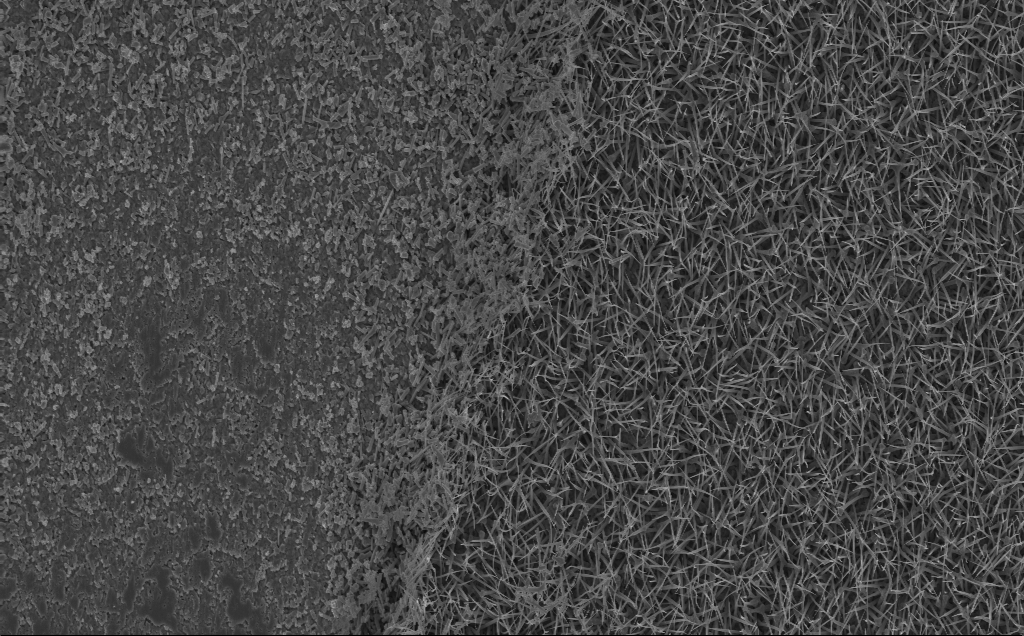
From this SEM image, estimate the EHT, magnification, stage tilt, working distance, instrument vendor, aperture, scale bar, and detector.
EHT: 5 kV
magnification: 6.1 K X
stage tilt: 0°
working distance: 5 mm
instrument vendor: Zeiss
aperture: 30 µm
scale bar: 10000 nm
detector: InLens